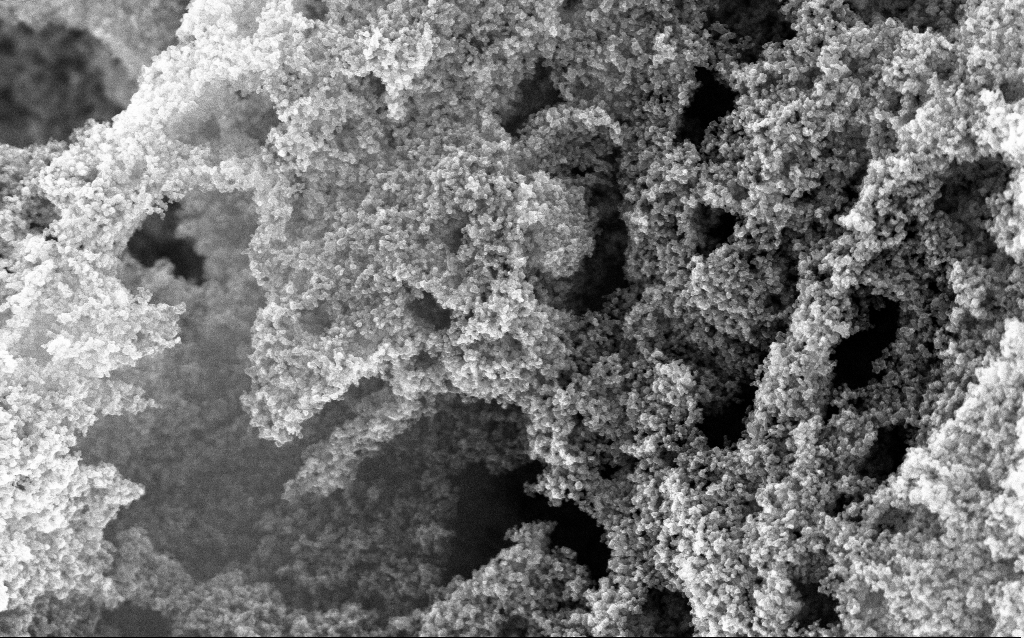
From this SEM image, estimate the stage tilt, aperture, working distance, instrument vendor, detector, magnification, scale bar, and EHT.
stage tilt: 0°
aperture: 30 µm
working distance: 2.4 mm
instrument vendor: Zeiss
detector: InLens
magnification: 65.04 K X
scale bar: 1000 nm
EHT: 10 kV